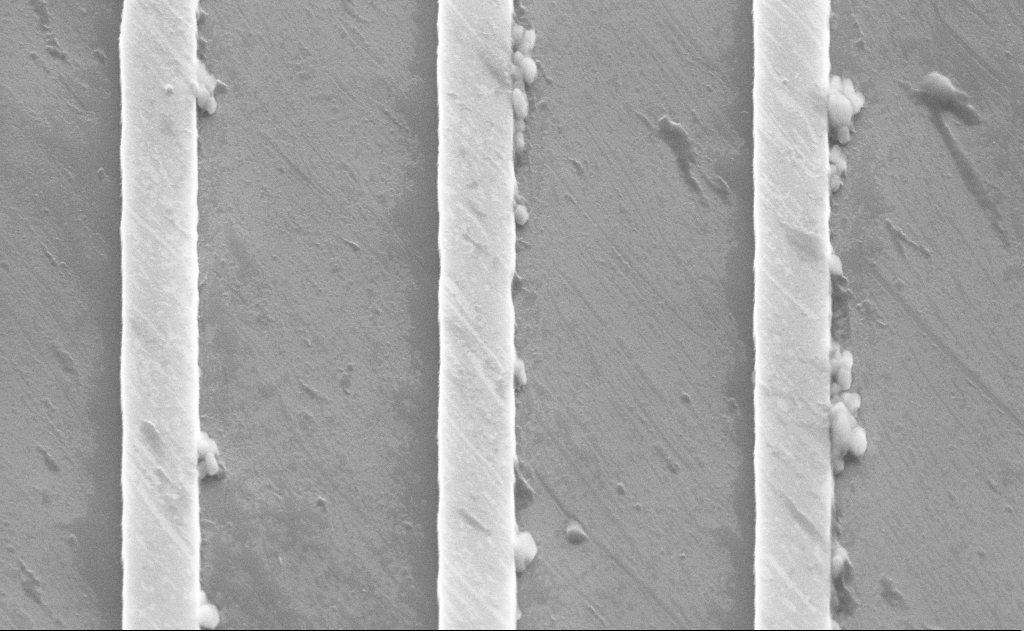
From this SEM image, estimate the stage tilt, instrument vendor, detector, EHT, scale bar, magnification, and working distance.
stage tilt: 50°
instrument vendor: Zeiss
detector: SE2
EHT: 10 kV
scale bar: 2000 nm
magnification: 28.85 K X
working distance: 15 mm